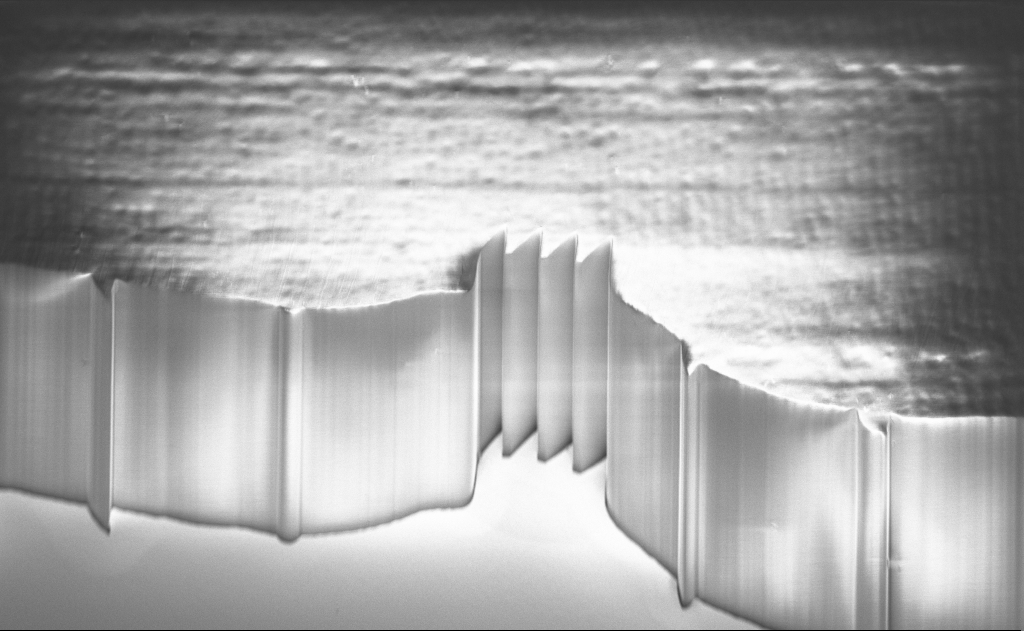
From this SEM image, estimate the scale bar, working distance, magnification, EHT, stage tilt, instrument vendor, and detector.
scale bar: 20000 nm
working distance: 8 mm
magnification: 1.12 K X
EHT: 1.5 kV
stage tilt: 45°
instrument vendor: Zeiss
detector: InLens